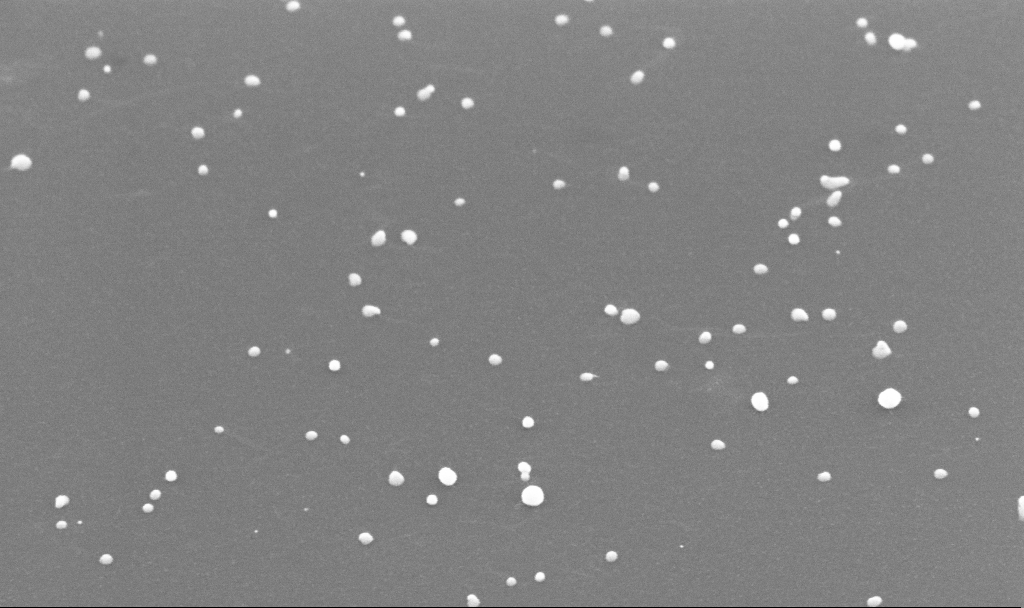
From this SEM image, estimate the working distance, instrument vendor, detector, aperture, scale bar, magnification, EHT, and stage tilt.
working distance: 4 mm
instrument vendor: Zeiss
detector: InLens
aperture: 30 µm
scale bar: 100 nm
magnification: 150 K X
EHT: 10 kV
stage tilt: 45°